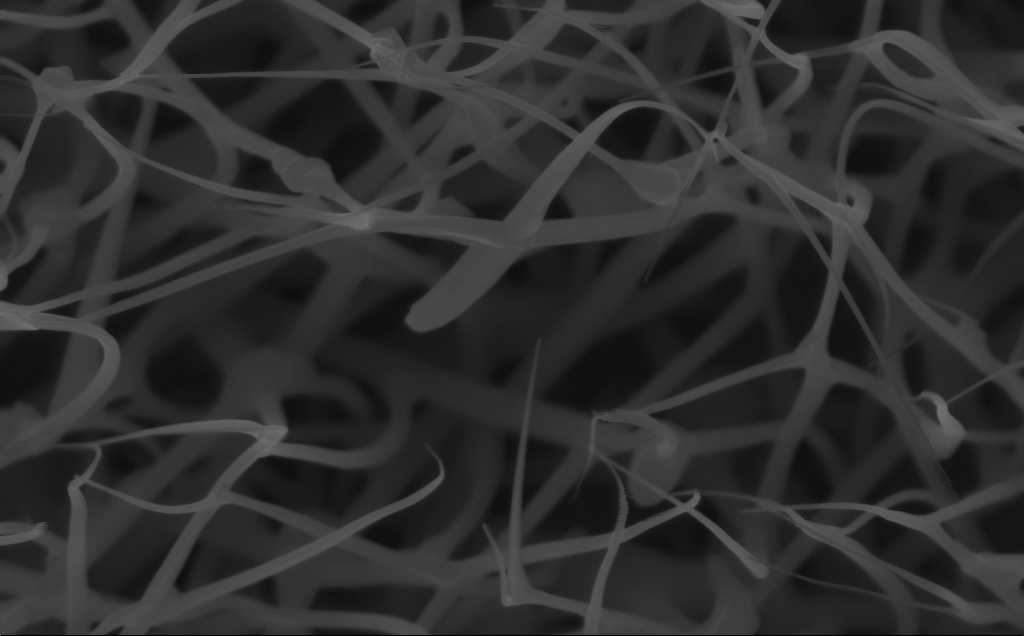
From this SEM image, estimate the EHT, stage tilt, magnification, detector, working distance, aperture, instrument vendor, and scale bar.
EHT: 10 kV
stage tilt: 0°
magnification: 80 K X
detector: InLens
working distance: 6 mm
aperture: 30 µm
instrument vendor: Zeiss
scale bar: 200 nm